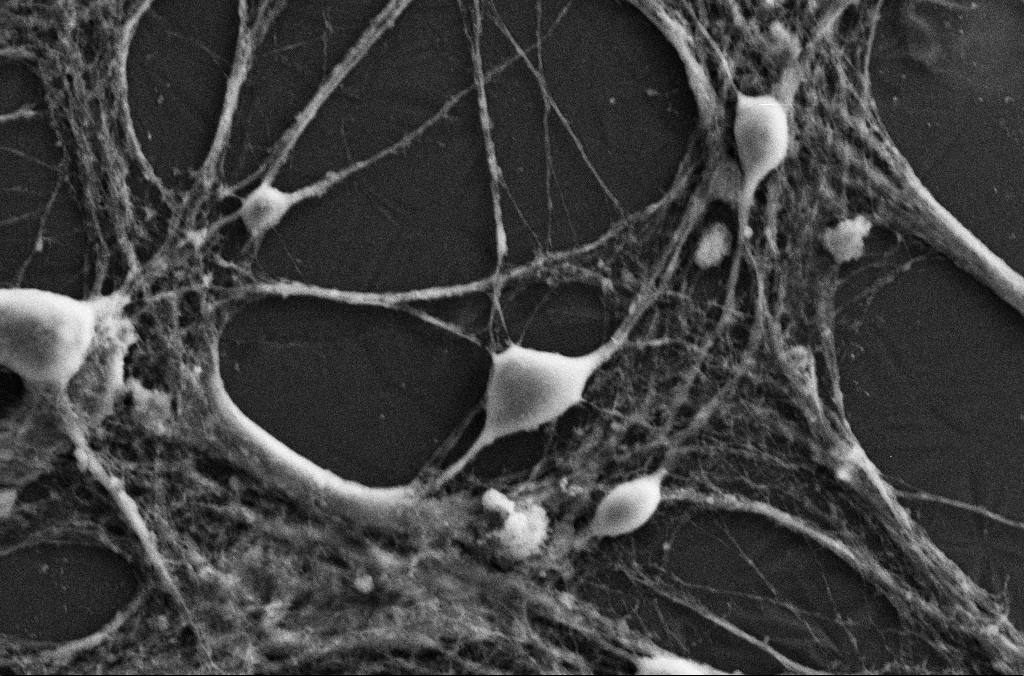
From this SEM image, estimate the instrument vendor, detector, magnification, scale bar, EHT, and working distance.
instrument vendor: Zeiss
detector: SE2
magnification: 2.5 K X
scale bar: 10000 nm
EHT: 15 kV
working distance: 3.1 mm